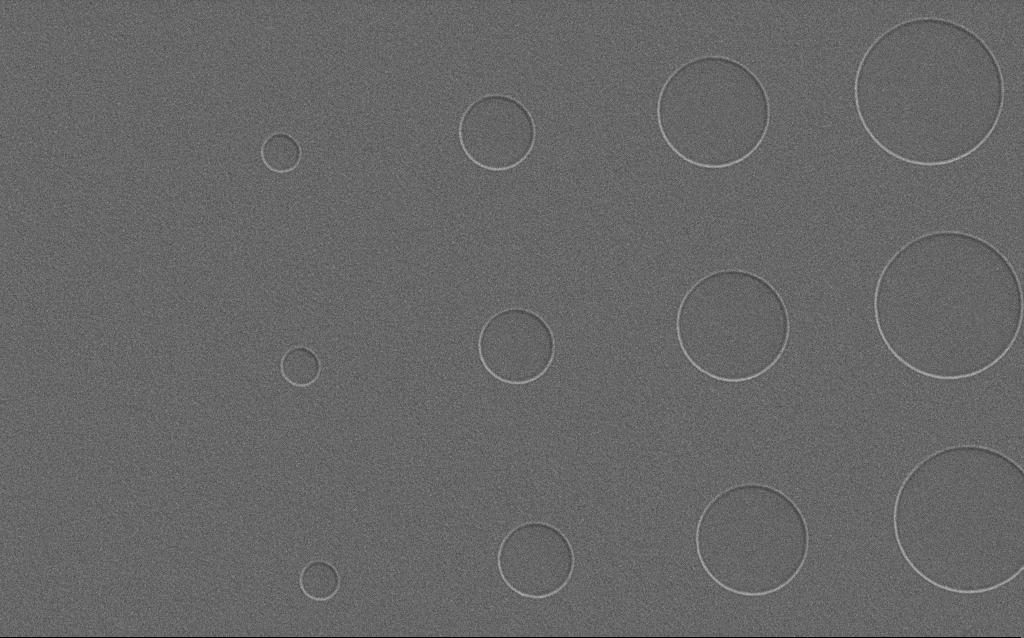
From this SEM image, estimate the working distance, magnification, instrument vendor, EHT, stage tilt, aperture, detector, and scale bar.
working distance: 5 mm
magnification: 13.19 K X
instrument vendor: Zeiss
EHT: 1 kV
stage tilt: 0°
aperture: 30 µm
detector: SE2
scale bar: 1000 nm